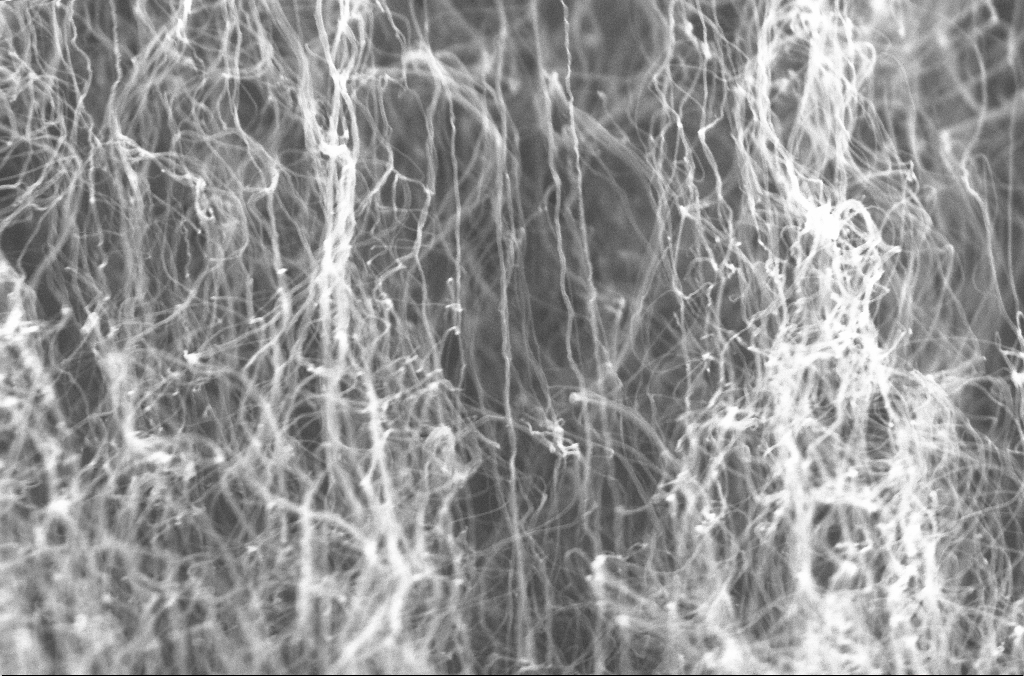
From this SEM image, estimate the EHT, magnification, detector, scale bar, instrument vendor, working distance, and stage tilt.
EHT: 20 kV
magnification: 100 K X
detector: InLens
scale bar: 200 nm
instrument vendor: Zeiss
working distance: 4 mm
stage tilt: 0°